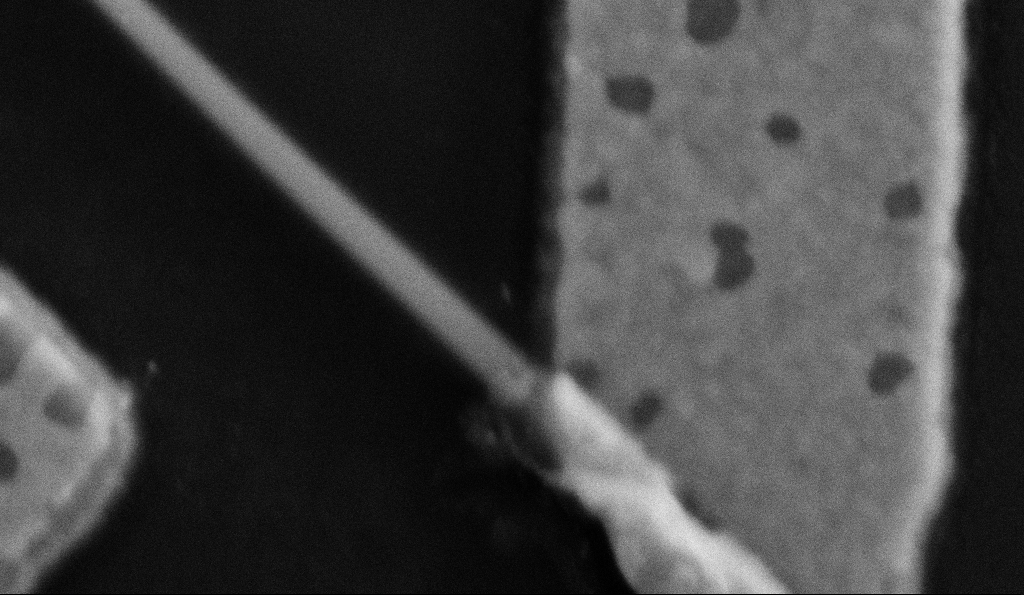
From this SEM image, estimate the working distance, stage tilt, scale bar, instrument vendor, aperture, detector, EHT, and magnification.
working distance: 8.5 mm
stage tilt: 0°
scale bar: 200 nm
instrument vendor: Zeiss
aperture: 30 µm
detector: SE2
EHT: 5 kV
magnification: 200 K X